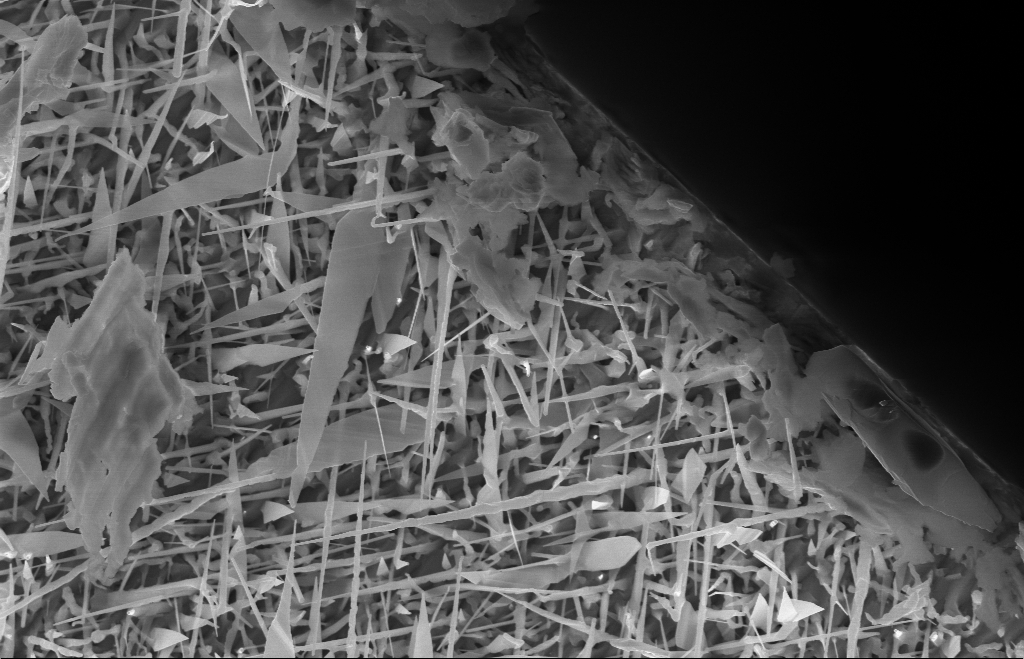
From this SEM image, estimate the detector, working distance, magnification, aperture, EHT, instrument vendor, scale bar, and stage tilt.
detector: InLens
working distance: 10 mm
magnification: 20 K X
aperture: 30 µm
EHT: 10 kV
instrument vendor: Zeiss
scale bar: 1000 nm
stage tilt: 0°